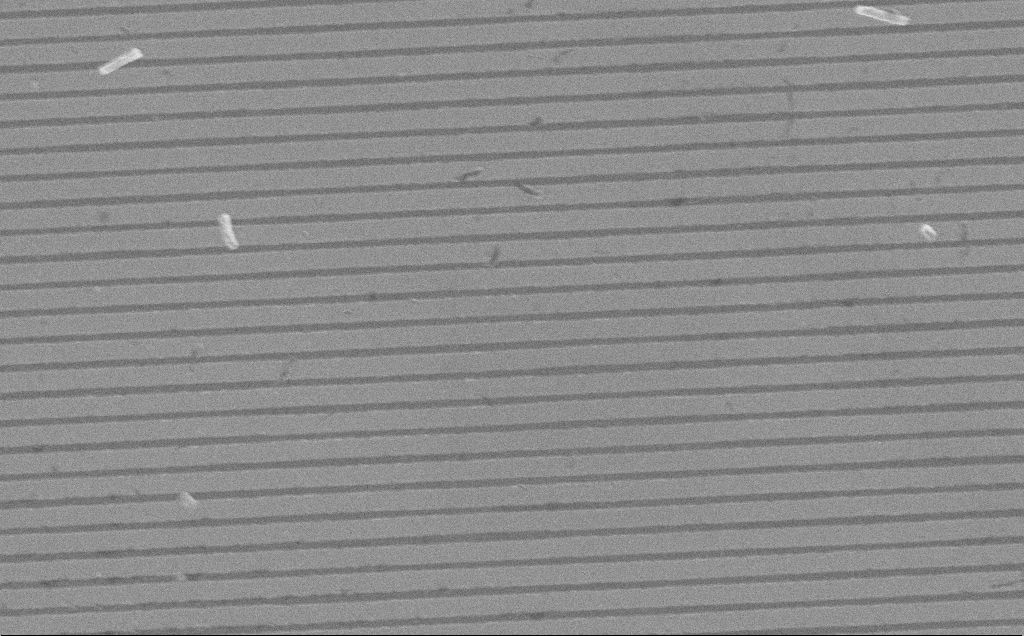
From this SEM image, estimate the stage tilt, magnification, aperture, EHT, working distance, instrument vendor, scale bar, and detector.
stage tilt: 0°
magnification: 40.08 K X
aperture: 30 µm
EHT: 10 kV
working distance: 7 mm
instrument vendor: Zeiss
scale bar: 1000 nm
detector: InLens